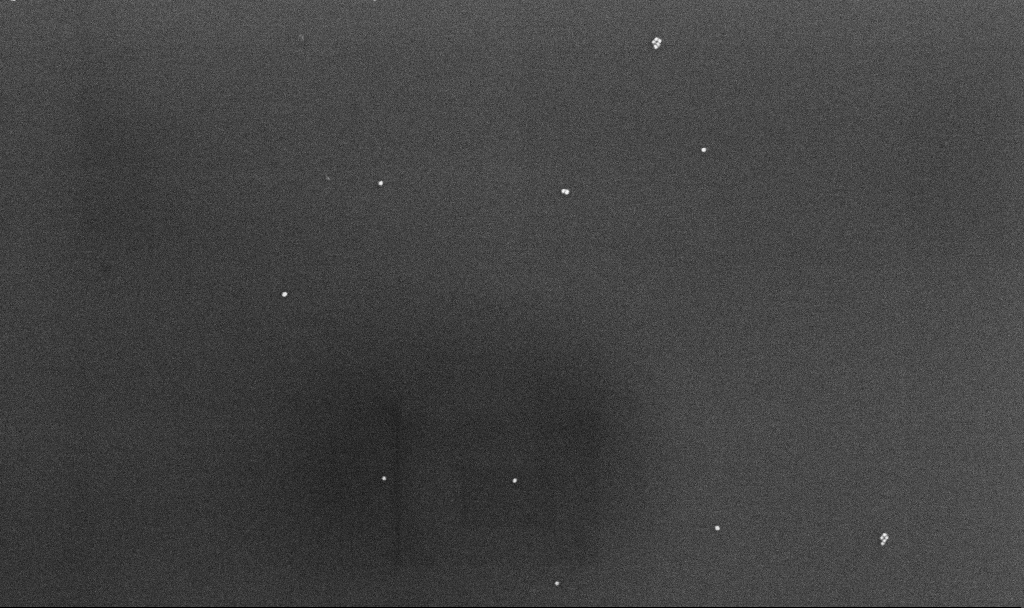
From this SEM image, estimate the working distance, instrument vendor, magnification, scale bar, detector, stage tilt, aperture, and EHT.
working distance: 4.3 mm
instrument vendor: Zeiss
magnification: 83.42 K X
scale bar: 200 nm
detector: InLens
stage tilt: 0°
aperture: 30 µm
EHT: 10 kV